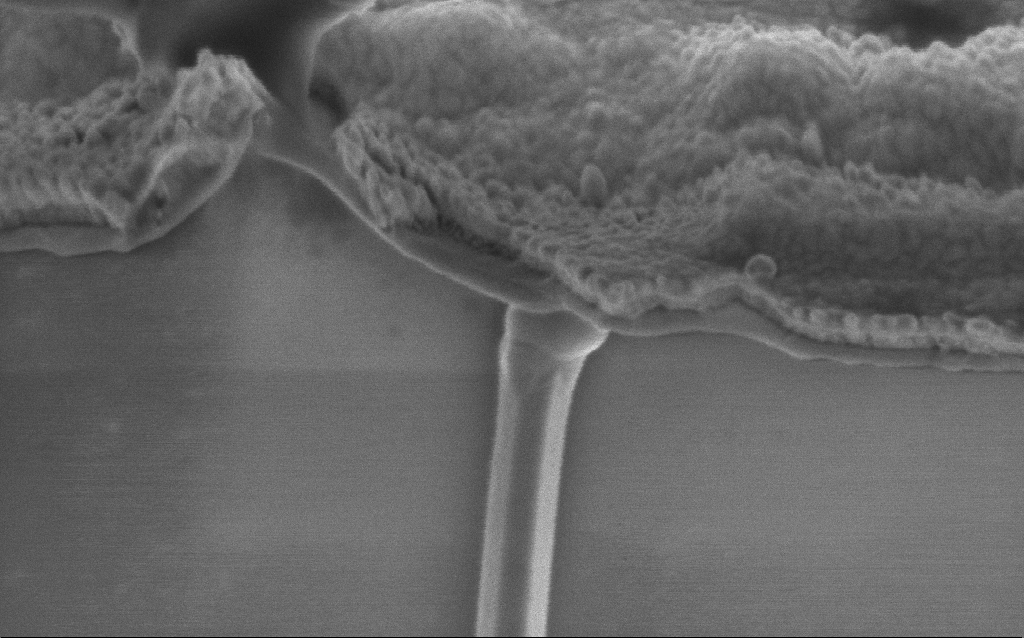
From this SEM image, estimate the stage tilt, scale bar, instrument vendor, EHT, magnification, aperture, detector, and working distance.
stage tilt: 0°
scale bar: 200 nm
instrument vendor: Zeiss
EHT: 2 kV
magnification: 133.46 K X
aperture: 30 µm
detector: InLens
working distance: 7.7 mm